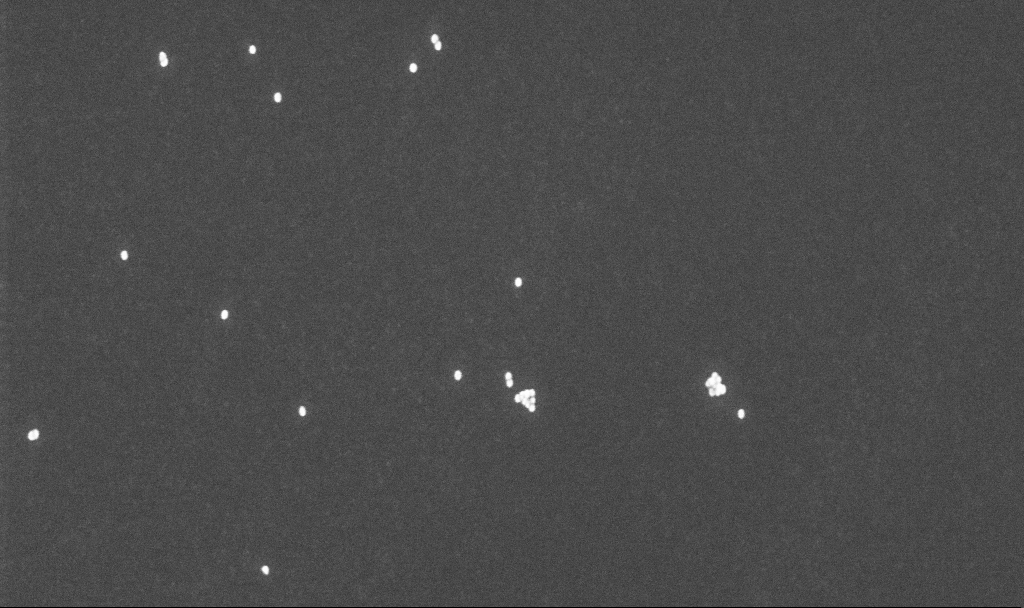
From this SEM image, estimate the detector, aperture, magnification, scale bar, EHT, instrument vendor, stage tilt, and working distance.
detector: InLens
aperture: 30 µm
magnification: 100 K X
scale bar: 200 nm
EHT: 10 kV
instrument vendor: Zeiss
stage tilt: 0°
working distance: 4.8 mm